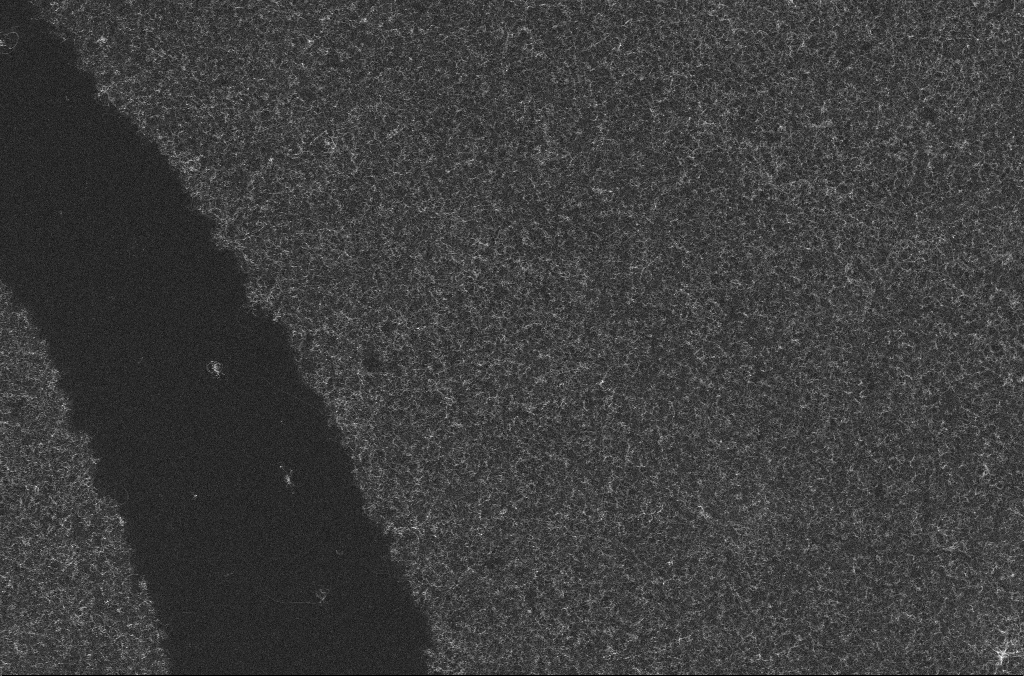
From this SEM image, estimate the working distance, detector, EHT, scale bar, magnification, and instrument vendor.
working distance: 3.2 mm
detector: InLens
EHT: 10 kV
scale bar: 10000 nm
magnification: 5 K X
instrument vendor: Zeiss